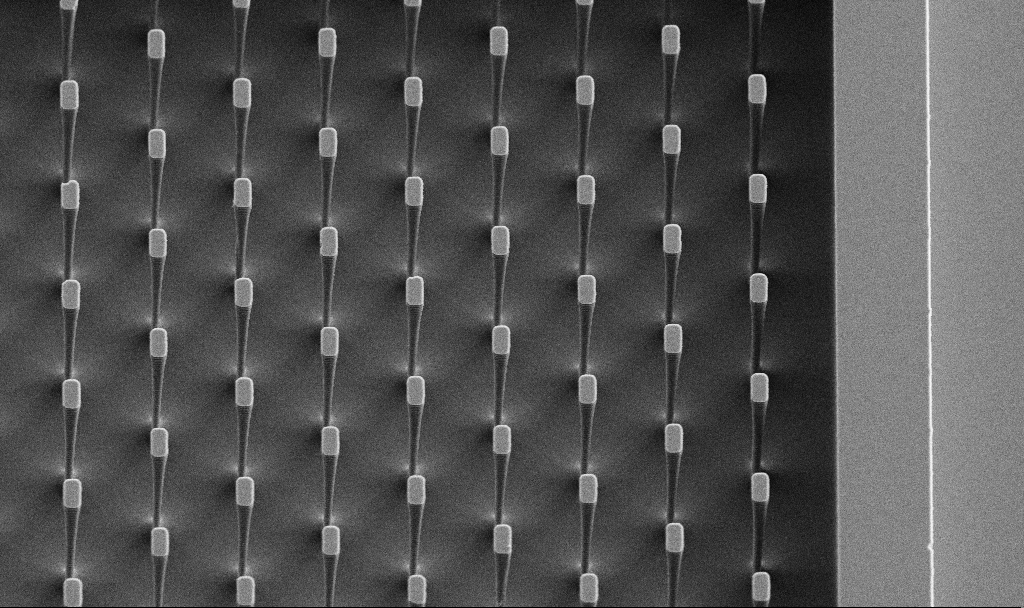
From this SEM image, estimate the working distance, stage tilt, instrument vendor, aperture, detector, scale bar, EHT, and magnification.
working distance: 6.8 mm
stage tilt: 29.2°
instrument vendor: Zeiss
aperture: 30 µm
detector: SE2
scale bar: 10000 nm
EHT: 5 kV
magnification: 3.53 K X